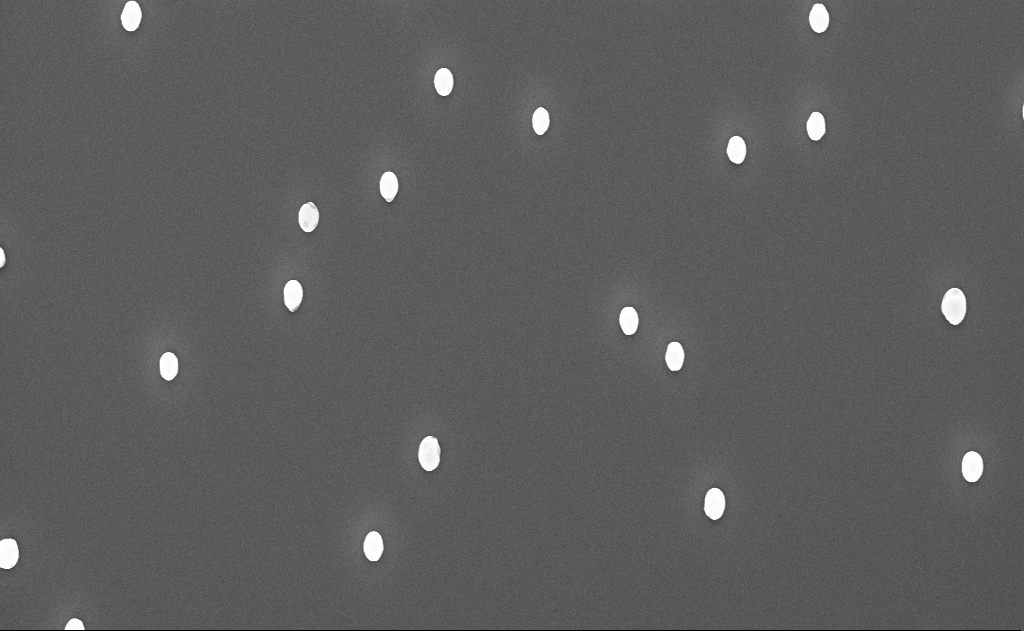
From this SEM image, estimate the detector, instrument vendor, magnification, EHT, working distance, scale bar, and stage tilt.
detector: InLens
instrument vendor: Zeiss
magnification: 20 K X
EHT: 10 kV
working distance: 12 mm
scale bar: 2000 nm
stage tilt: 0°